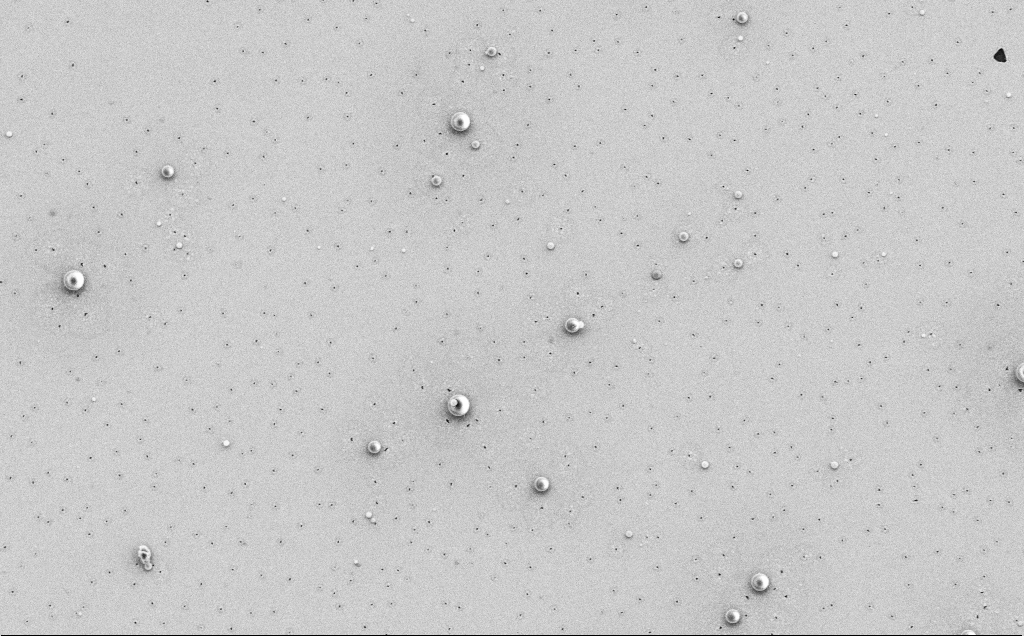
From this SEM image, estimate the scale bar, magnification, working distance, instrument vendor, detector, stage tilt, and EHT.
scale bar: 10000 nm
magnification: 4.2 K X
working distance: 12 mm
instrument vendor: Zeiss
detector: SE2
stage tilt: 0°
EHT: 5 kV